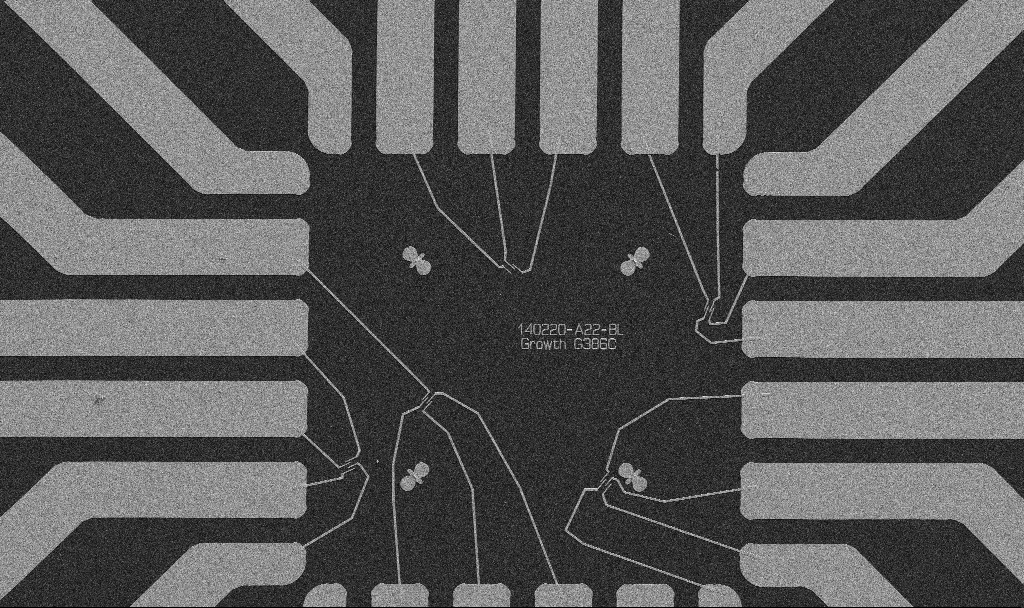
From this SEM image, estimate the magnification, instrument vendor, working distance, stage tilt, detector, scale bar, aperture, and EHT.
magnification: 1 K X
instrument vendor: Zeiss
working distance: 10.7 mm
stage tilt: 0°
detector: SE2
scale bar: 20000 nm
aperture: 30 µm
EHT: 5 kV